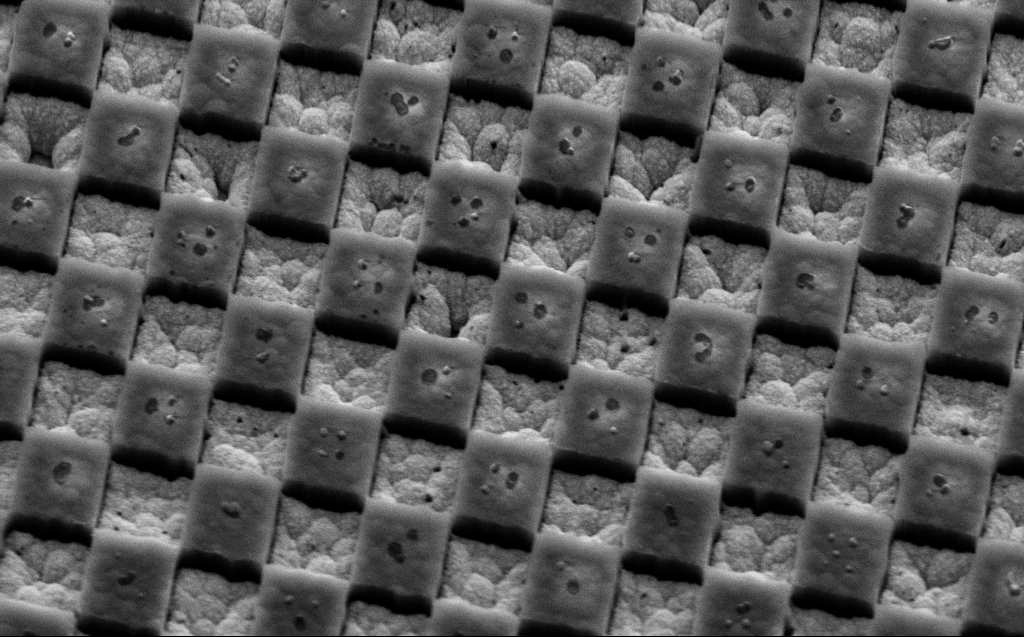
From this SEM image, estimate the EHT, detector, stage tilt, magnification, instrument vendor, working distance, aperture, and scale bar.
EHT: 5 kV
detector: SE2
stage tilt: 45°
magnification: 31.87 K X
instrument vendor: Zeiss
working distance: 9 mm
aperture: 30 µm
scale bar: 2000 nm